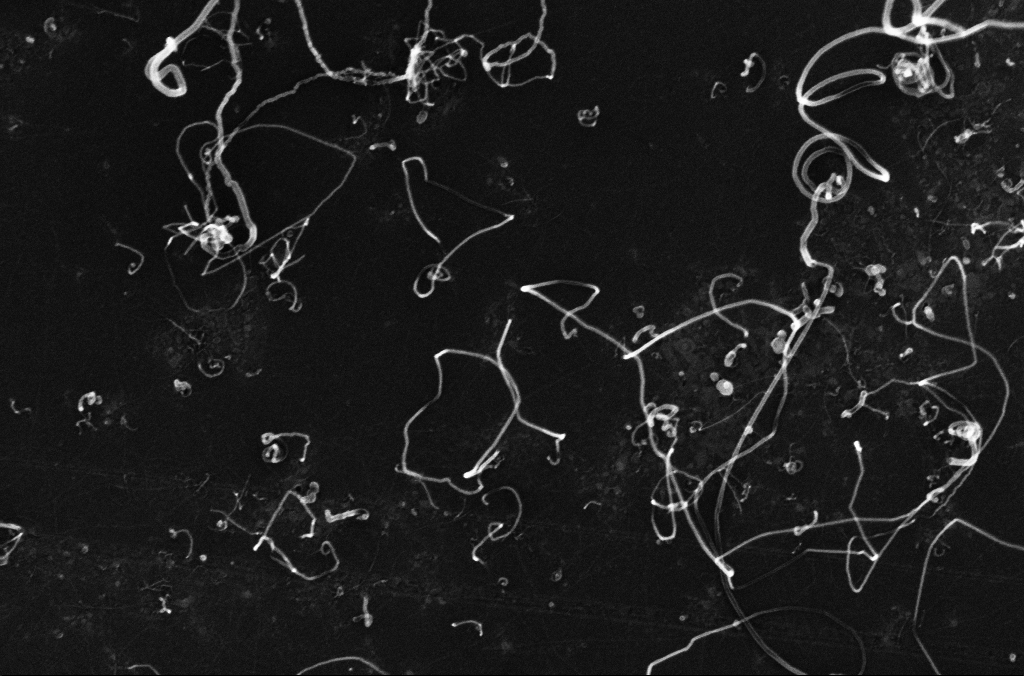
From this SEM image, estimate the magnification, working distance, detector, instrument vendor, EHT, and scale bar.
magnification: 100 K X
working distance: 3.3 mm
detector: InLens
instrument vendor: Zeiss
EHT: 10 kV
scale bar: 200 nm